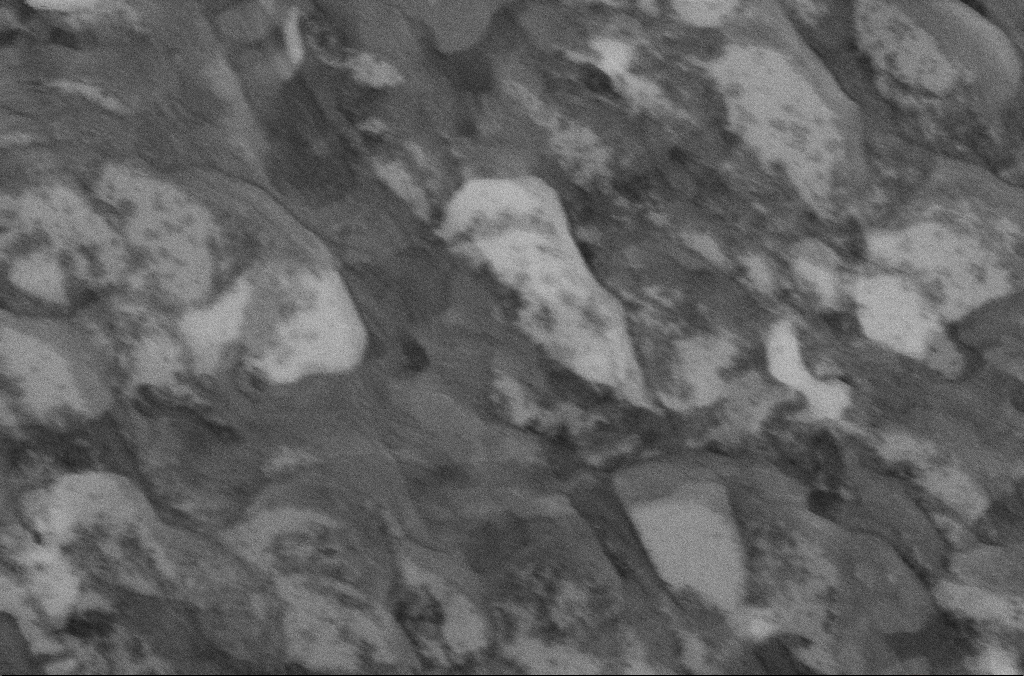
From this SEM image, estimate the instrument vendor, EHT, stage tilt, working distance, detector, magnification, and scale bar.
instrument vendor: Zeiss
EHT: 5 kV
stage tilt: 0°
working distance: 6.7 mm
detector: InLens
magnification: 70 K X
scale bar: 200 nm